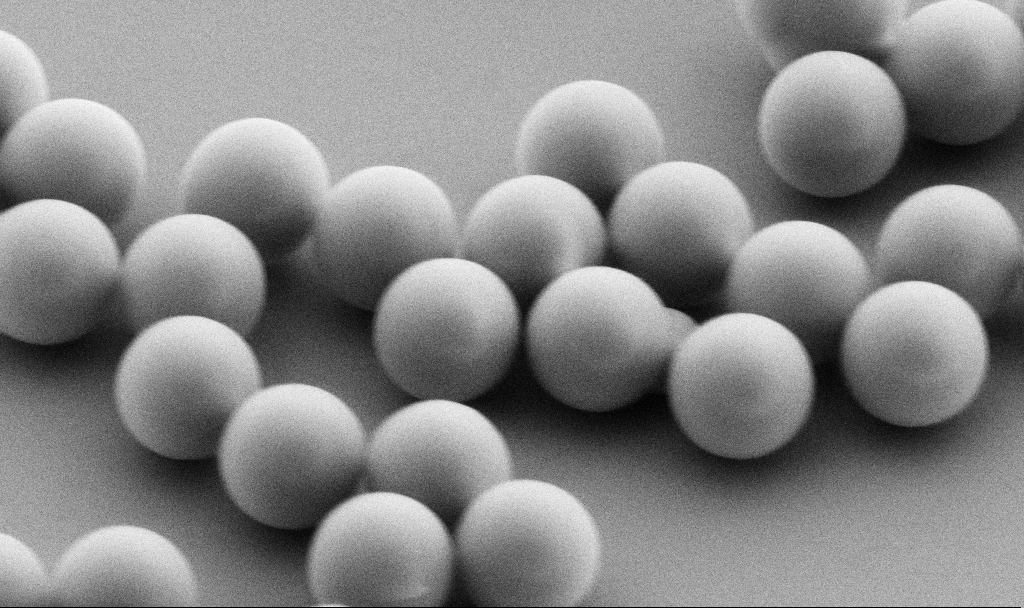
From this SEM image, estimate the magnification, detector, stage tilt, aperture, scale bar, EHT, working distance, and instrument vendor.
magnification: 41.91 K X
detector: SE2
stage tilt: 45°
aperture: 30 µm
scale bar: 1000 nm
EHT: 5 kV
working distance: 8 mm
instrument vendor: Zeiss